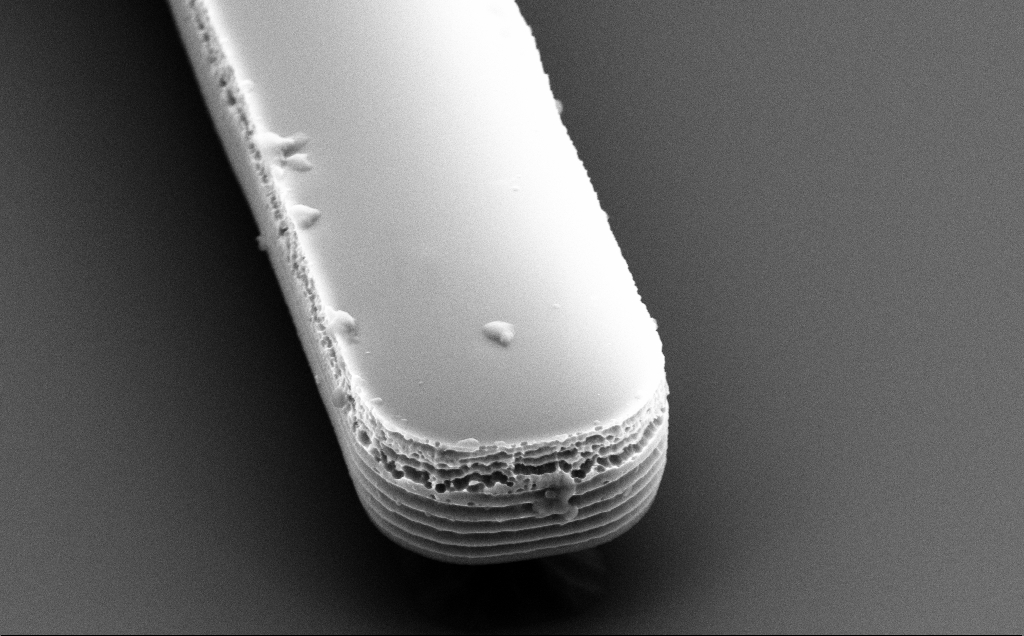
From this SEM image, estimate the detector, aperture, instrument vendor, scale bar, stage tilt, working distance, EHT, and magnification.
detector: SE2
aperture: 30 µm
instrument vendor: Zeiss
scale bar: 1000 nm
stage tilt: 50°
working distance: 10 mm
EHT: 5 kV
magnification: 22.54 K X